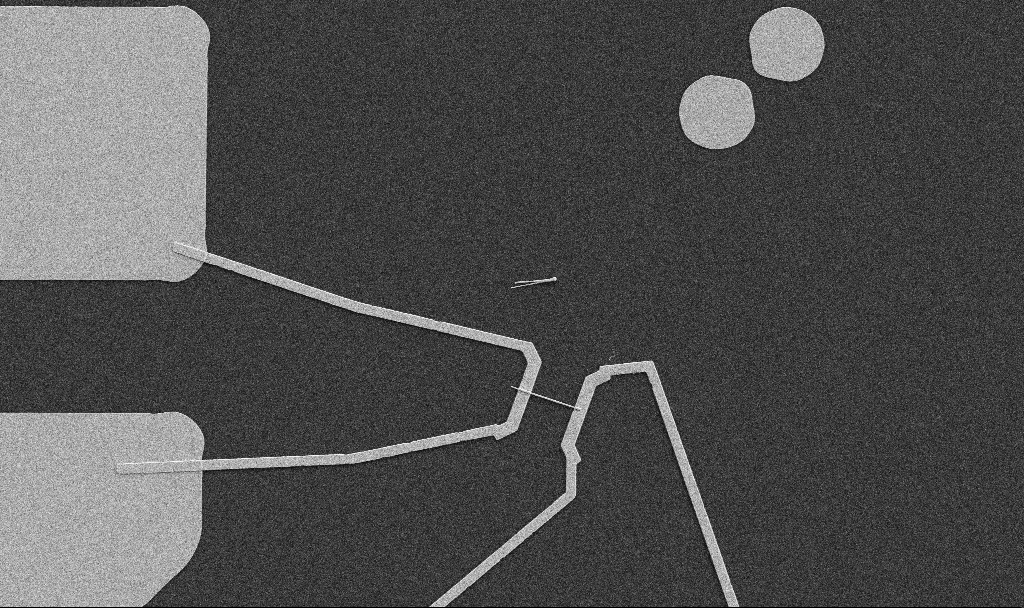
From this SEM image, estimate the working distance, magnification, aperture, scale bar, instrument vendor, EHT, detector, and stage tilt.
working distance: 10.7 mm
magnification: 5 K X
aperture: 30 µm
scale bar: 10000 nm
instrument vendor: Zeiss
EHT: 5 kV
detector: SE2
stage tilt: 0°